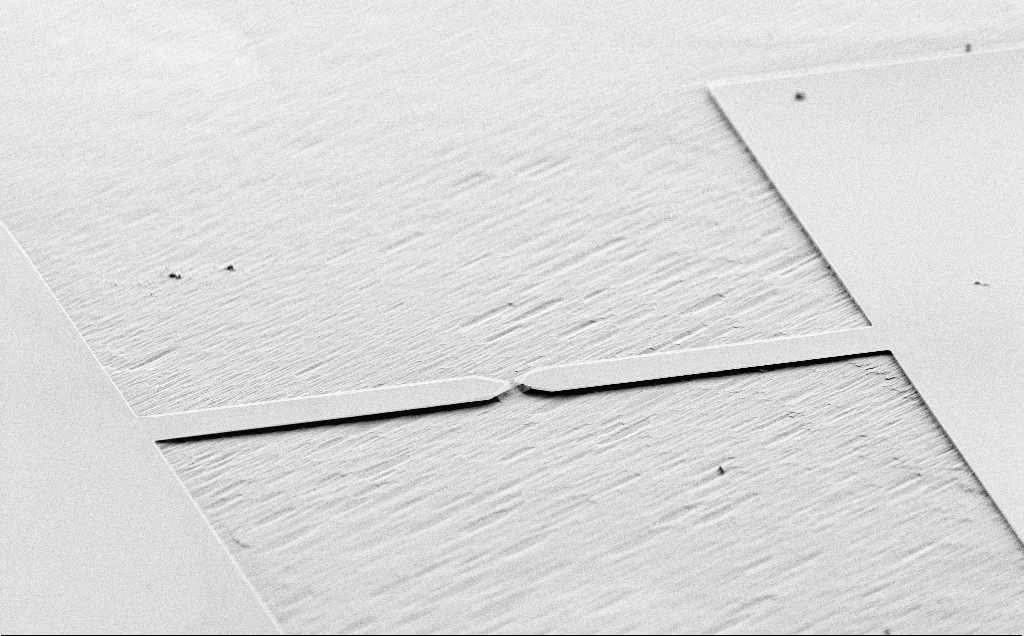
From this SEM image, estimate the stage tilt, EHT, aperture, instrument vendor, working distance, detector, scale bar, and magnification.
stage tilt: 70°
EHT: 5 kV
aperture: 30 µm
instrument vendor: Zeiss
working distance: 7 mm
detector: SE2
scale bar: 20000 nm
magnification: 1.18 K X